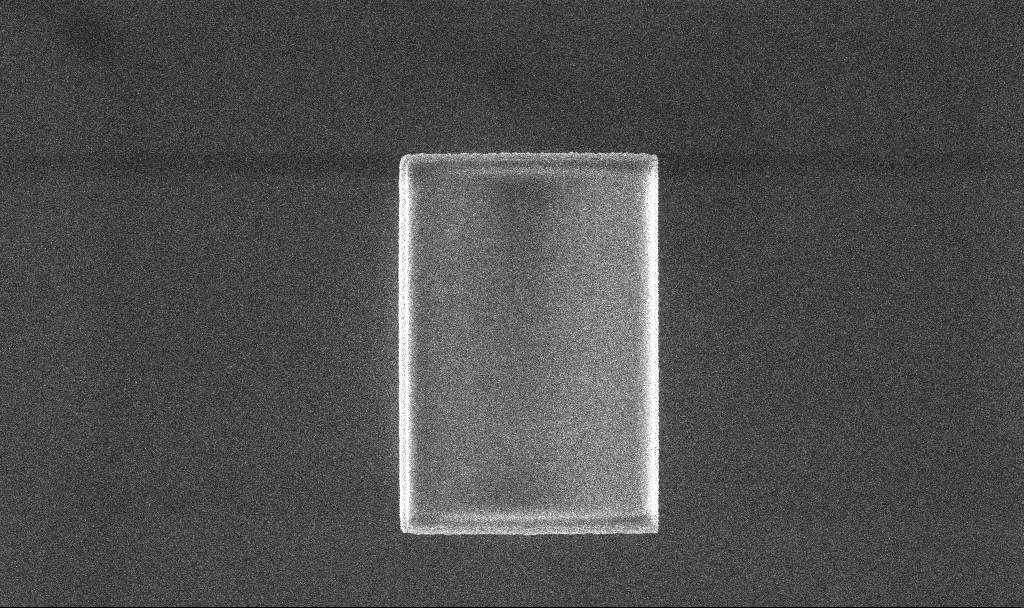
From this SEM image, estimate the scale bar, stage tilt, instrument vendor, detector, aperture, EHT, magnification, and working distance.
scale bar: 1000 nm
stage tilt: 0°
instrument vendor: Zeiss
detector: InLens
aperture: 30 µm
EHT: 5 kV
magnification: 31.42 K X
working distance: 3.3 mm